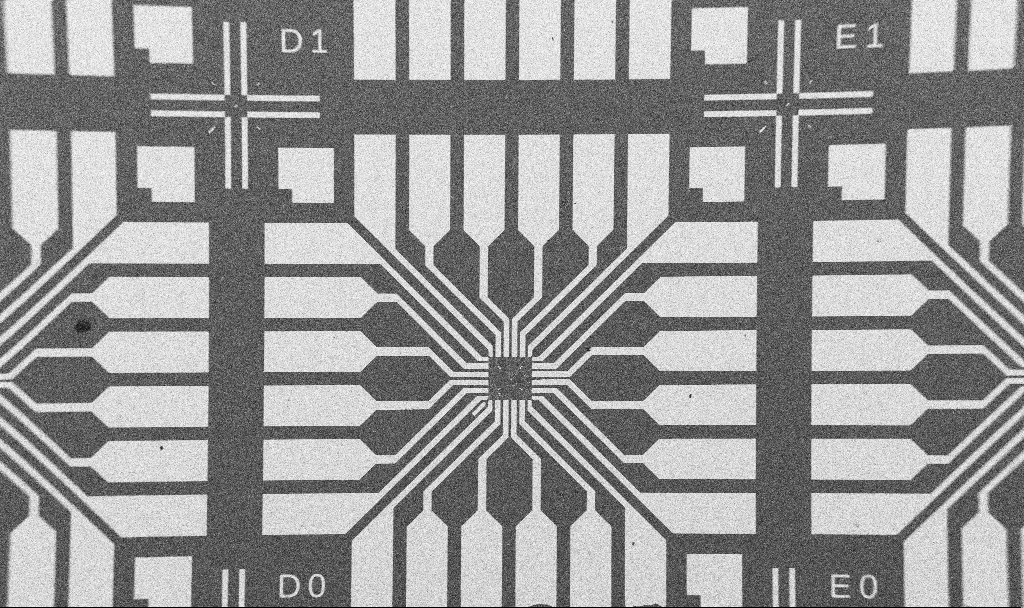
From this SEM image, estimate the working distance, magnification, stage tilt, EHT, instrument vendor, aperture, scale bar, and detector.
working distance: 10.7 mm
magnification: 0.1 K X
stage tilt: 0°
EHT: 5 kV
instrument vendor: Zeiss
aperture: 30 µm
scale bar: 200000 nm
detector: SE2